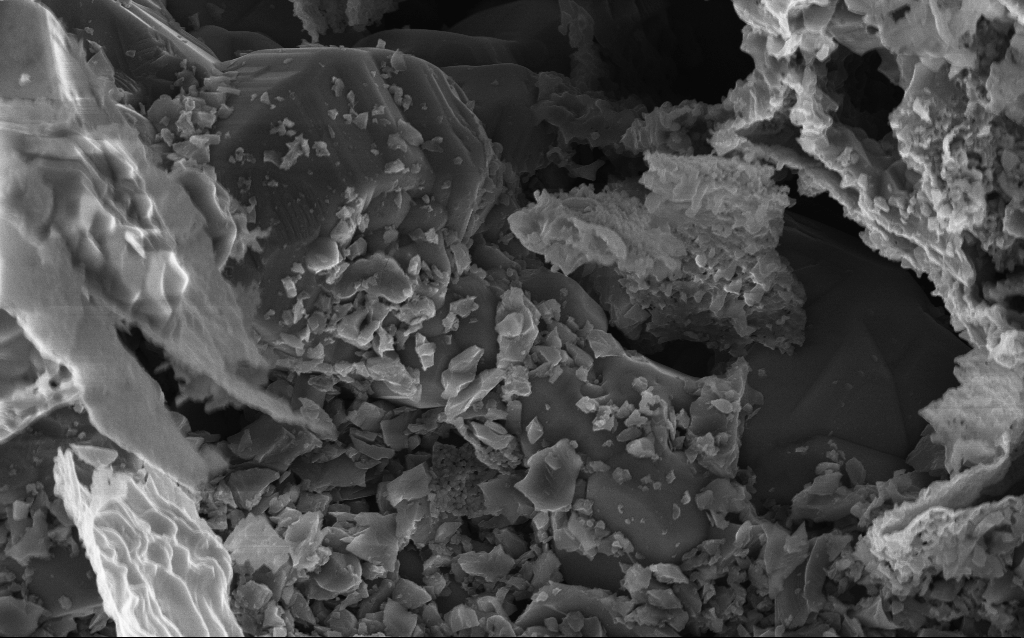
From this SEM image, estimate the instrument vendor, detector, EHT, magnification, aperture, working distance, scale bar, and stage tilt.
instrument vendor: Zeiss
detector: InLens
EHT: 10 kV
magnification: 10 K X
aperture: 30 µm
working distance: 3 mm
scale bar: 2000 nm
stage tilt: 0°